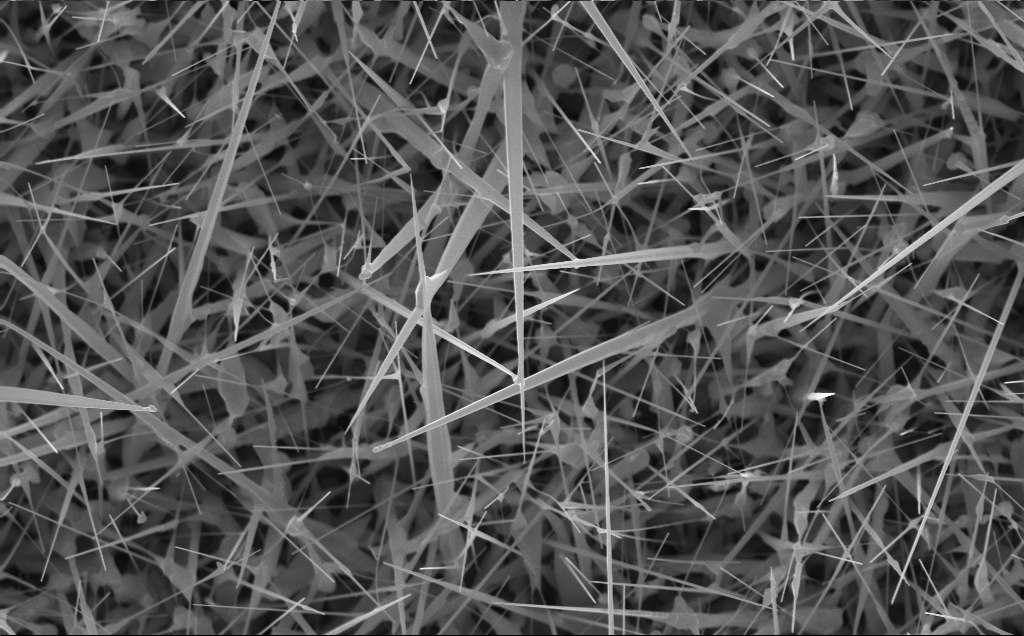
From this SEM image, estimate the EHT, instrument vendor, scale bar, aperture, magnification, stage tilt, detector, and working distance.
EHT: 10 kV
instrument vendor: Zeiss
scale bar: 2000 nm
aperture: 30 µm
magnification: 20 K X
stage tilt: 0°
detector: InLens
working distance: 4 mm